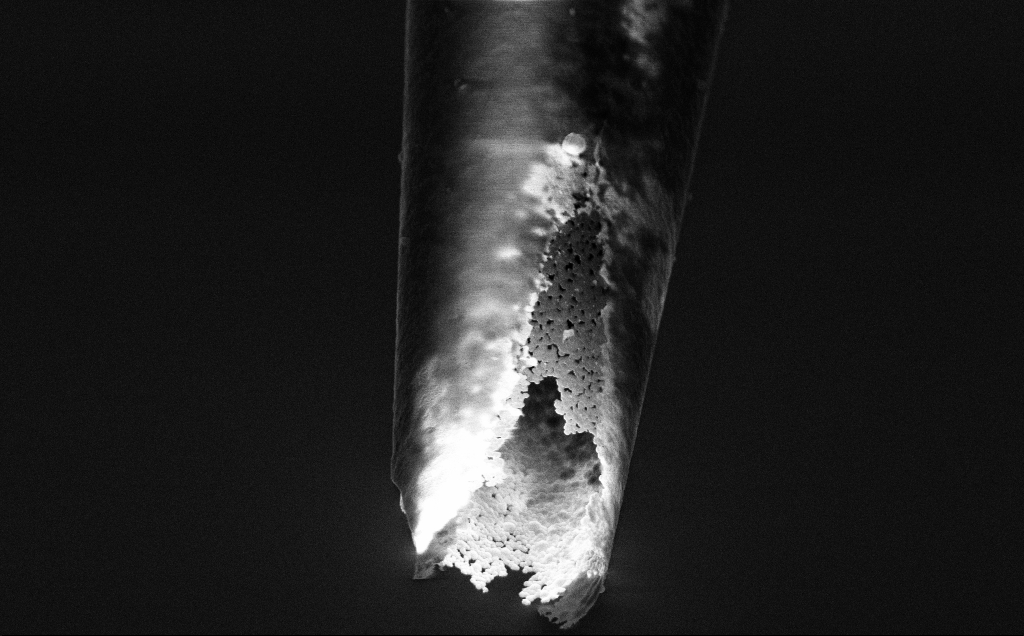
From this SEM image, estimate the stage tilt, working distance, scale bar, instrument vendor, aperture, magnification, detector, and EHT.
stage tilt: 45°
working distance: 7.4 mm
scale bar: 1000 nm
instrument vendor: Zeiss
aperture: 30 µm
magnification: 25 K X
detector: InLens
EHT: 3 kV